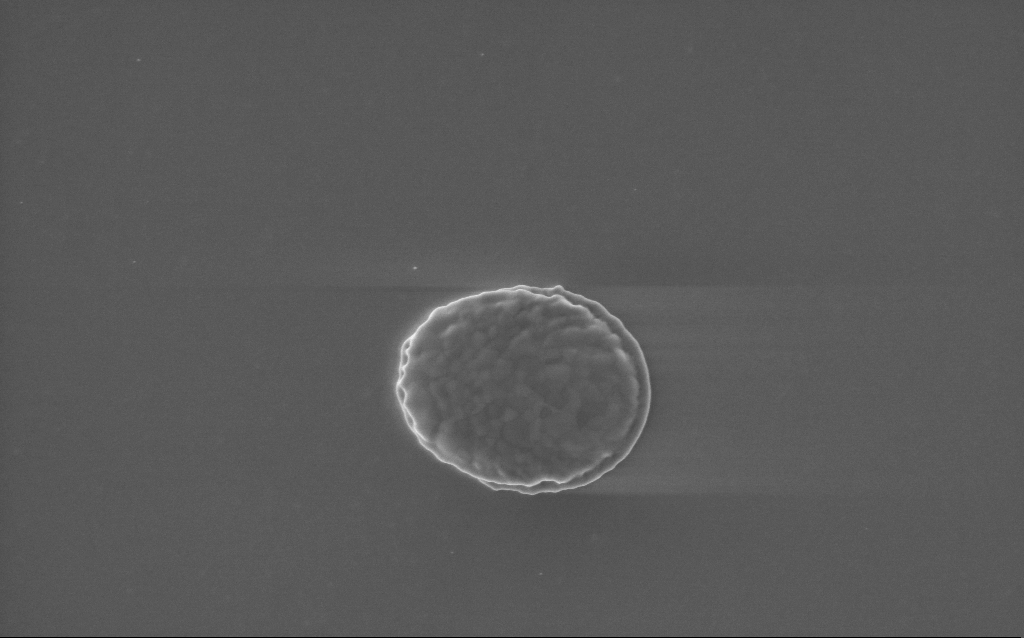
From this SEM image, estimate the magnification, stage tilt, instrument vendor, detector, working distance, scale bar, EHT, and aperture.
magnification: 28.66 K X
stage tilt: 0°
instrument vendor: Zeiss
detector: InLens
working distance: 2 mm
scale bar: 2000 nm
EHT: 5 kV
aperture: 30 µm